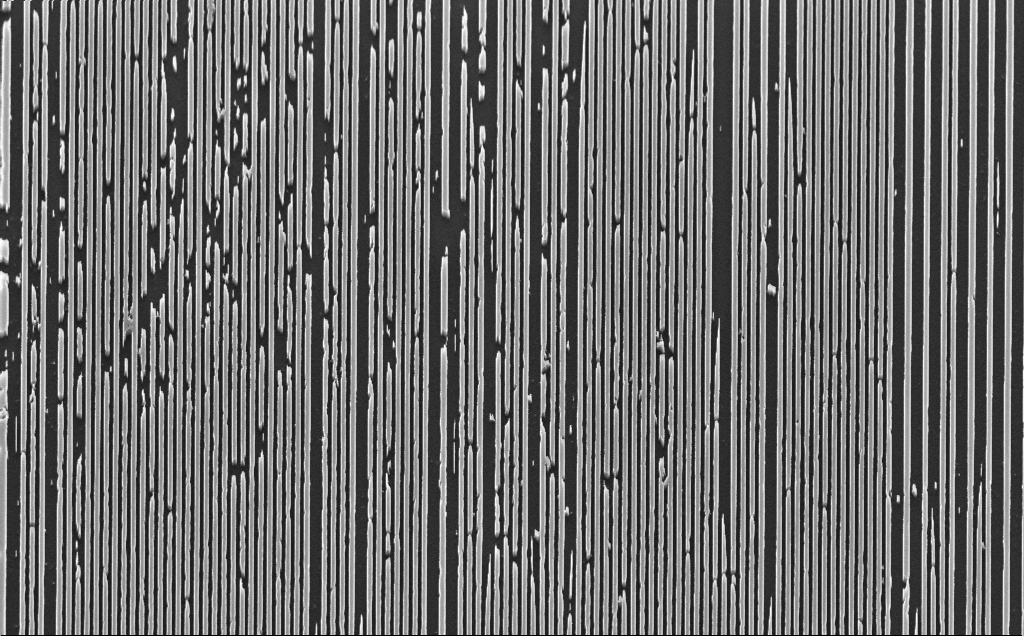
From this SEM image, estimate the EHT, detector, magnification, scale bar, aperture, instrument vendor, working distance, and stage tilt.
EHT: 10 kV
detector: InLens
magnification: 16.8 K X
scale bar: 2000 nm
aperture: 30 µm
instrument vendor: Zeiss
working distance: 5 mm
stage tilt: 30°